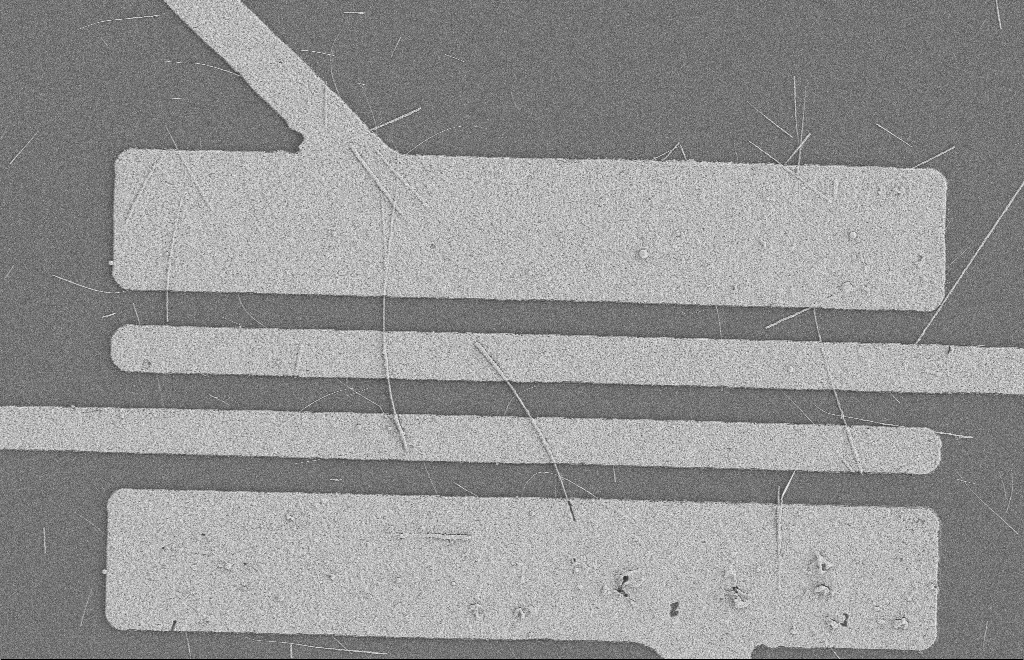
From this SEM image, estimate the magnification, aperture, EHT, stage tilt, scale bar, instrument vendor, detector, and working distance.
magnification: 5.01 K X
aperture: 20 µm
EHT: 2 kV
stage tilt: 0°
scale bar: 2000 nm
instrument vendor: Zeiss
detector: SE2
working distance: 8 mm